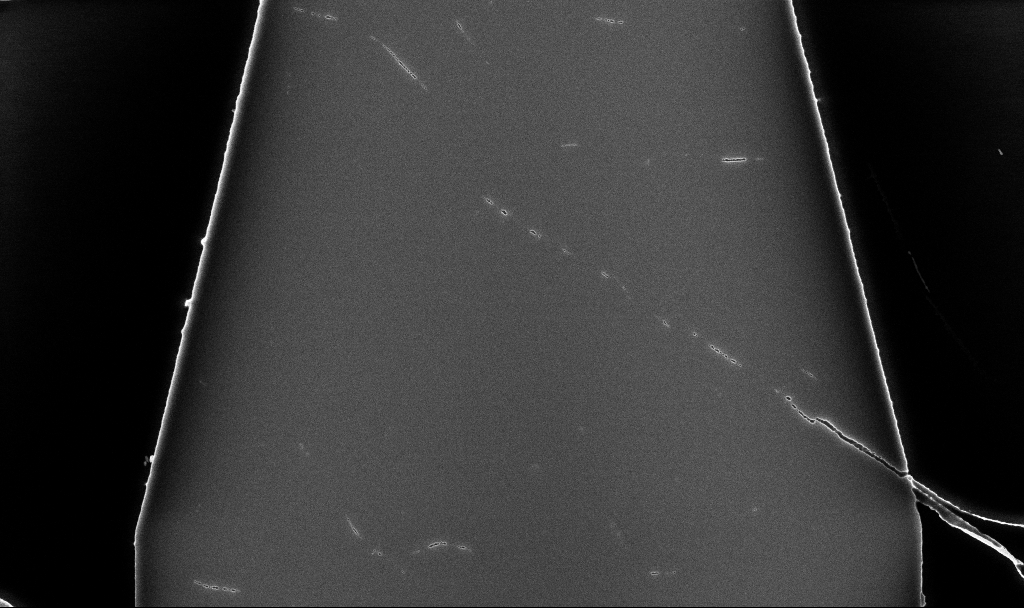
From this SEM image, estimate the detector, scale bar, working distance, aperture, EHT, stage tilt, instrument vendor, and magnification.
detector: InLens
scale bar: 2000 nm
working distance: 5.2 mm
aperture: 30 µm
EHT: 5 kV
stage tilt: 0°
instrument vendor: Zeiss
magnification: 14.72 K X